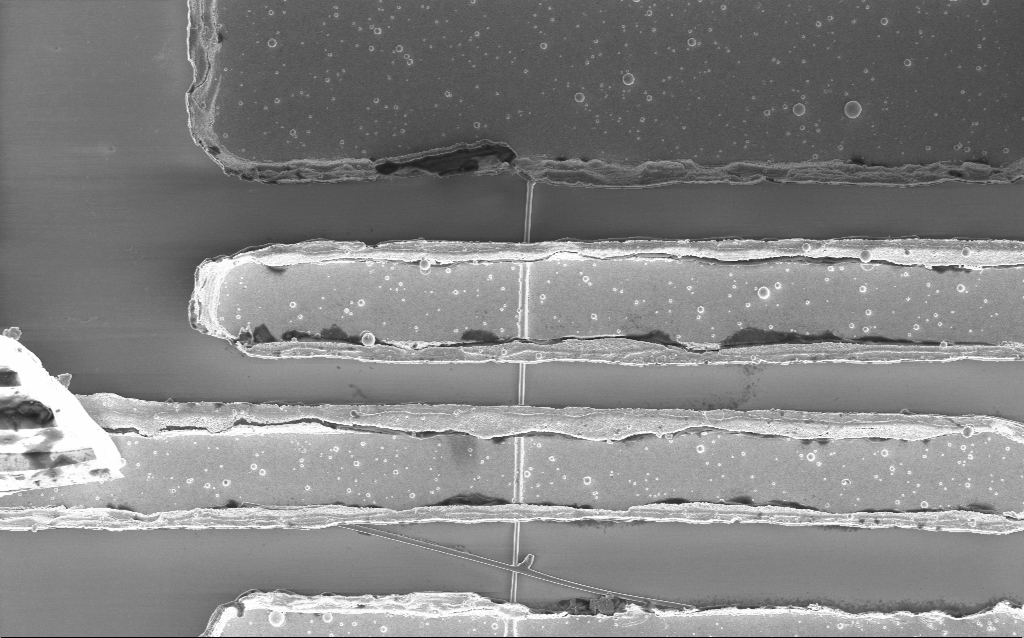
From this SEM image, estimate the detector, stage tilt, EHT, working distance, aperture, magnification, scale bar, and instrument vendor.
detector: InLens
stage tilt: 0°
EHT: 2 kV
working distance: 7.7 mm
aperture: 30 µm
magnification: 10.56 K X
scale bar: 2000 nm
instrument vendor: Zeiss